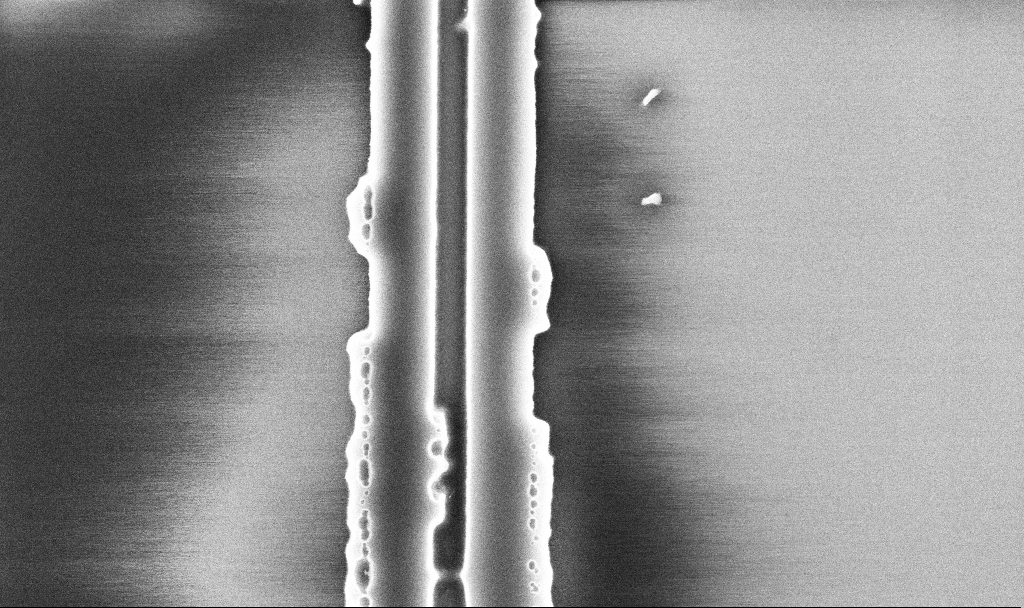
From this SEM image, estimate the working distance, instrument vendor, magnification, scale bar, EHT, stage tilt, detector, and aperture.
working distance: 10.1 mm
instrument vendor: Zeiss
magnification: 54.54 K X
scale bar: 1000 nm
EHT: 5 kV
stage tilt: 0°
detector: InLens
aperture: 30 µm